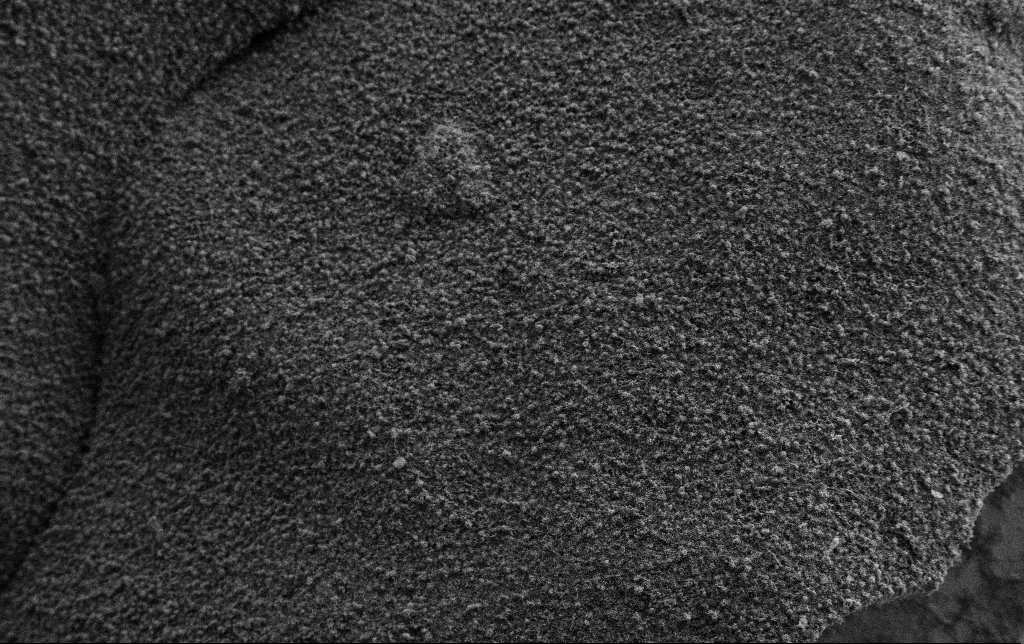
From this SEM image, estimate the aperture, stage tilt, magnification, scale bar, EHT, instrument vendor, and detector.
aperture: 30 µm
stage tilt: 0°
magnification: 0.5 K X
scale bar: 100000 nm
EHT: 2 kV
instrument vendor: Zeiss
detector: SE2